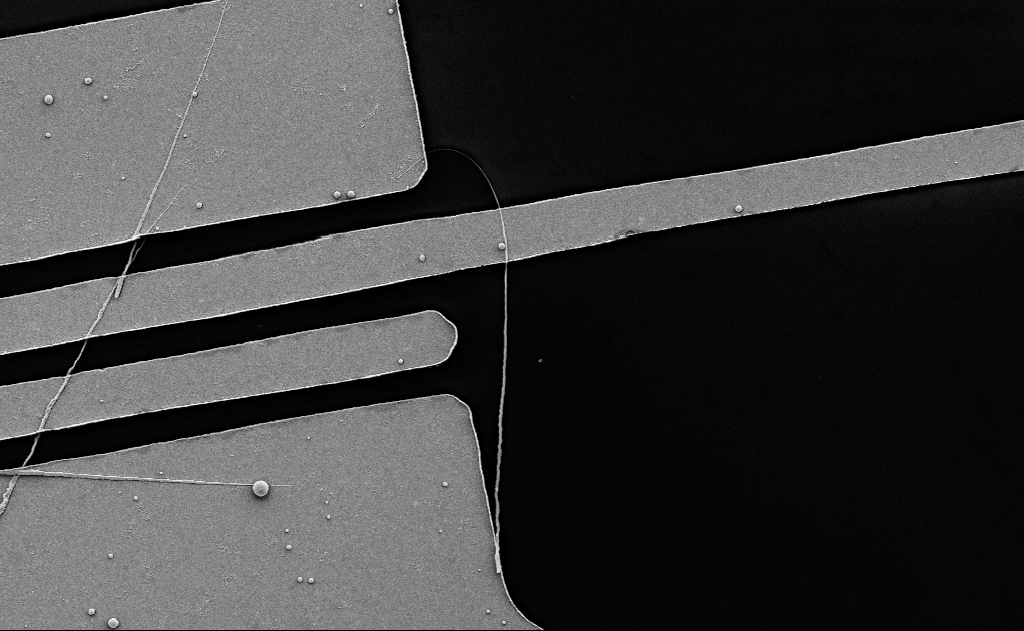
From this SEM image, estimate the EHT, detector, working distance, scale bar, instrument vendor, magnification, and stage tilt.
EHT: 5 kV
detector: SE2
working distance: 18 mm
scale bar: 2000 nm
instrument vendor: Zeiss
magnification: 7.98 K X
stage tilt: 0°